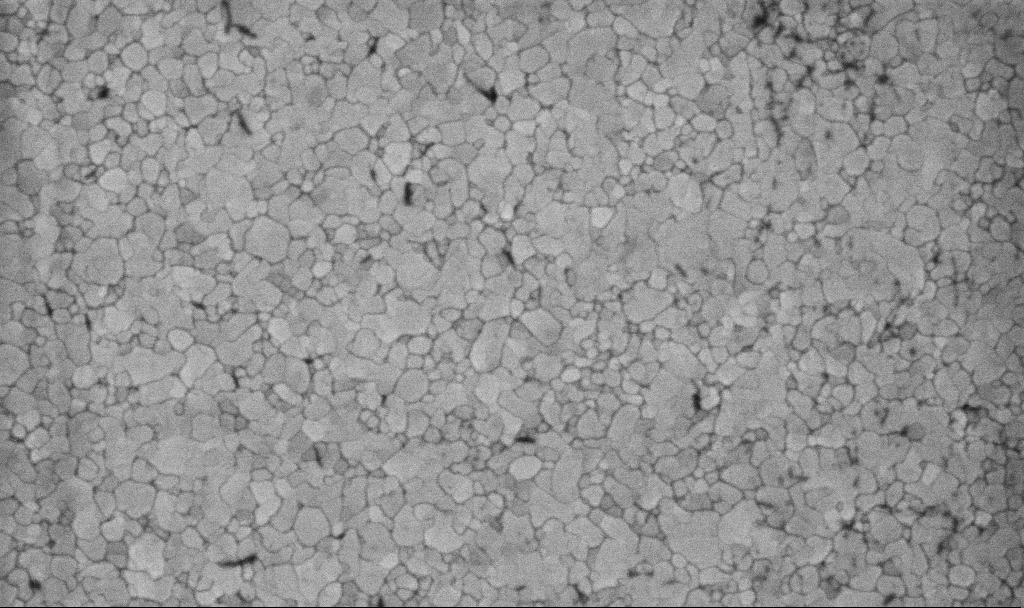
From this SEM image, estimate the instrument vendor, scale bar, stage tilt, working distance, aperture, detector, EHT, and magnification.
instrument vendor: Zeiss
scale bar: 200 nm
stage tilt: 0°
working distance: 3.1 mm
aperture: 30 µm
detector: InLens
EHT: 5 kV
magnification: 100.15 K X